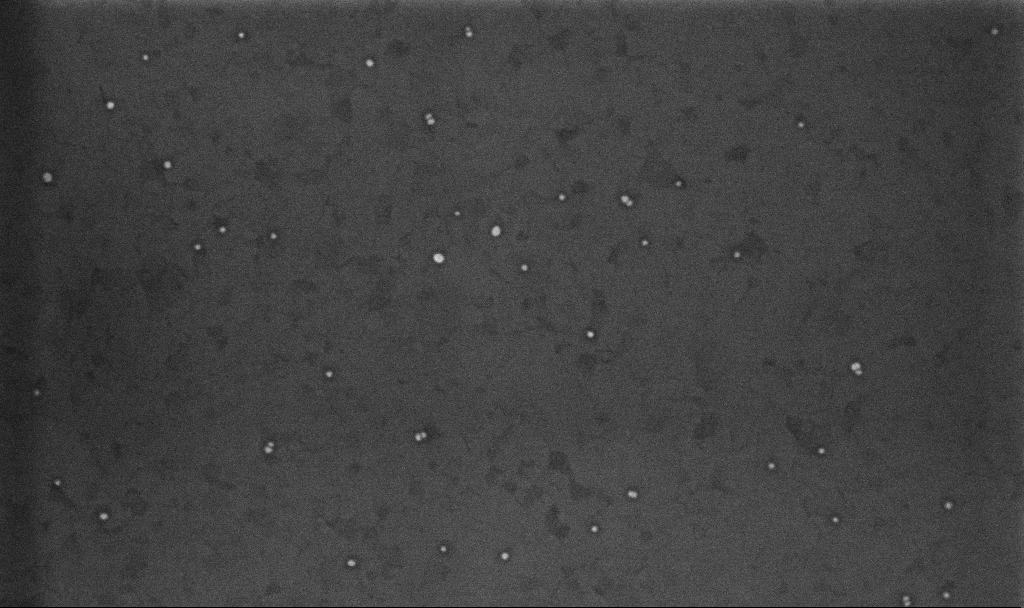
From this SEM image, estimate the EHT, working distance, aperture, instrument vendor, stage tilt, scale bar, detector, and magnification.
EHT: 10 kV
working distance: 3.3 mm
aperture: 30 µm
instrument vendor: Zeiss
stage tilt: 0°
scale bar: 200 nm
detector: InLens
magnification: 100.38 K X